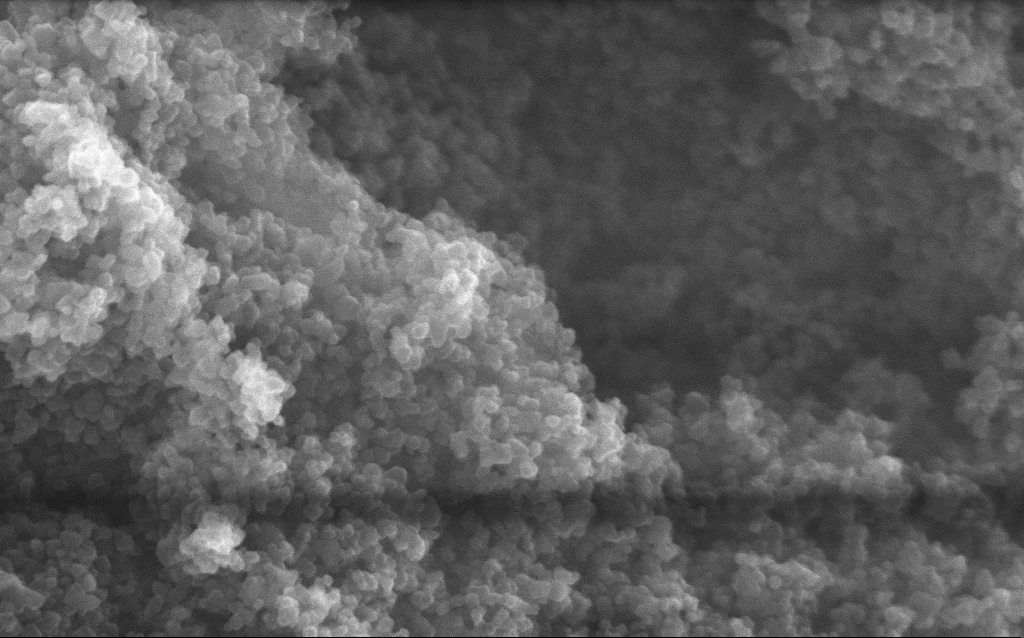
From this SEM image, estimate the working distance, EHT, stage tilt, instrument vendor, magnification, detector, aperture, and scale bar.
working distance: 2.7 mm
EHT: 10 kV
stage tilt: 0°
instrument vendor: Zeiss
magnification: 204.13 K X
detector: InLens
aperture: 30 µm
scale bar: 100 nm